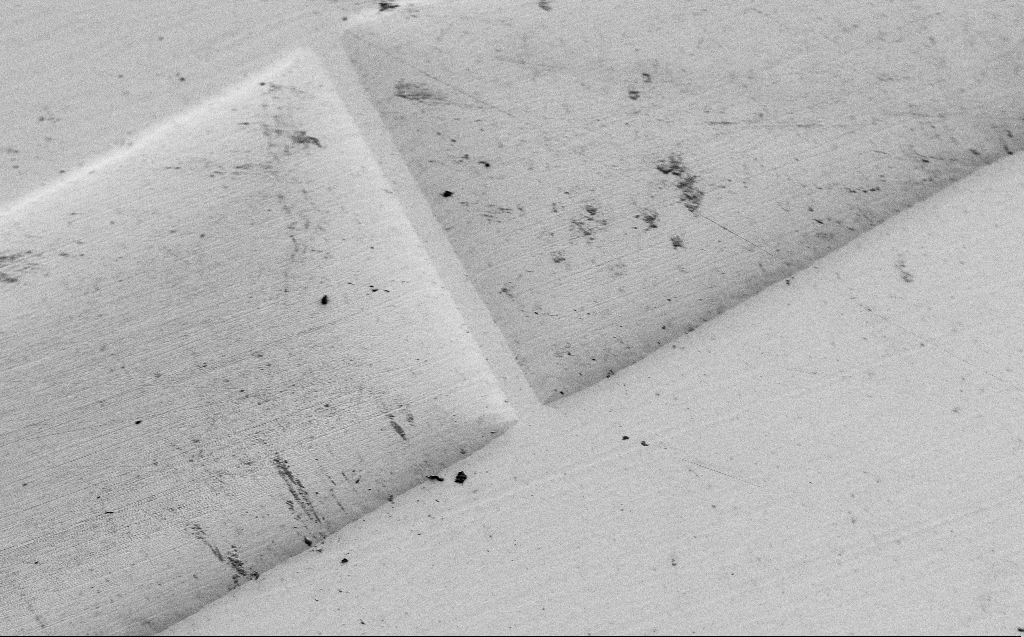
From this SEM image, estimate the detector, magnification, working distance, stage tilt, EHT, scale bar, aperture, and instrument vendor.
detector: SE2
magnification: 1.24 K X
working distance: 7 mm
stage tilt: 45°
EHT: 1.2 kV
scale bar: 20000 nm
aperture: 30 µm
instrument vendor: Zeiss